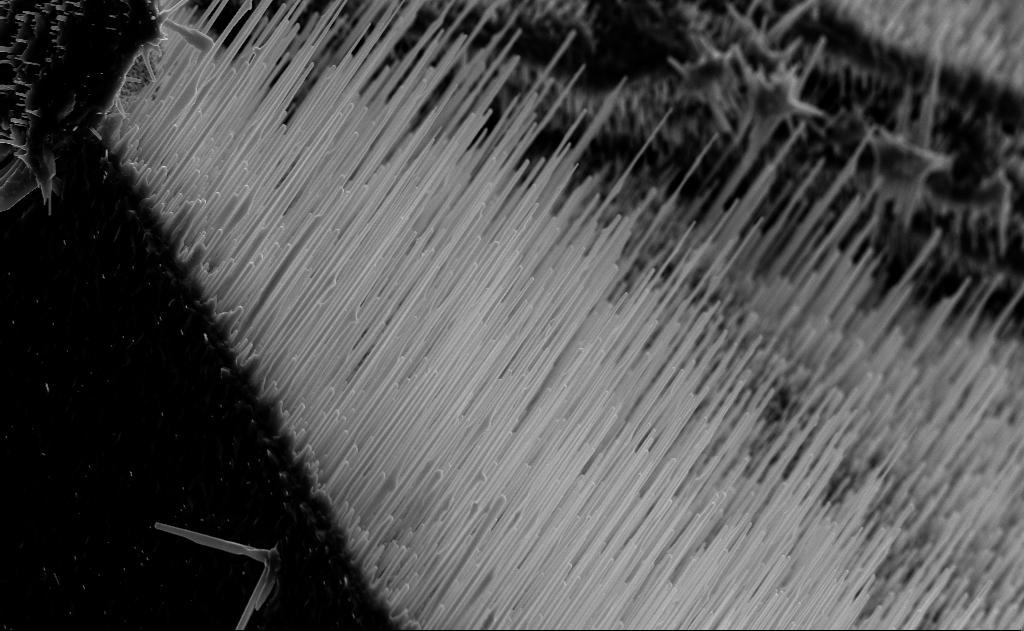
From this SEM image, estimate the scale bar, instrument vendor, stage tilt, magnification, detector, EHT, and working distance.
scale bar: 2000 nm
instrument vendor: Zeiss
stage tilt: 0°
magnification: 10 K X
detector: InLens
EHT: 10 kV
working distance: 6 mm